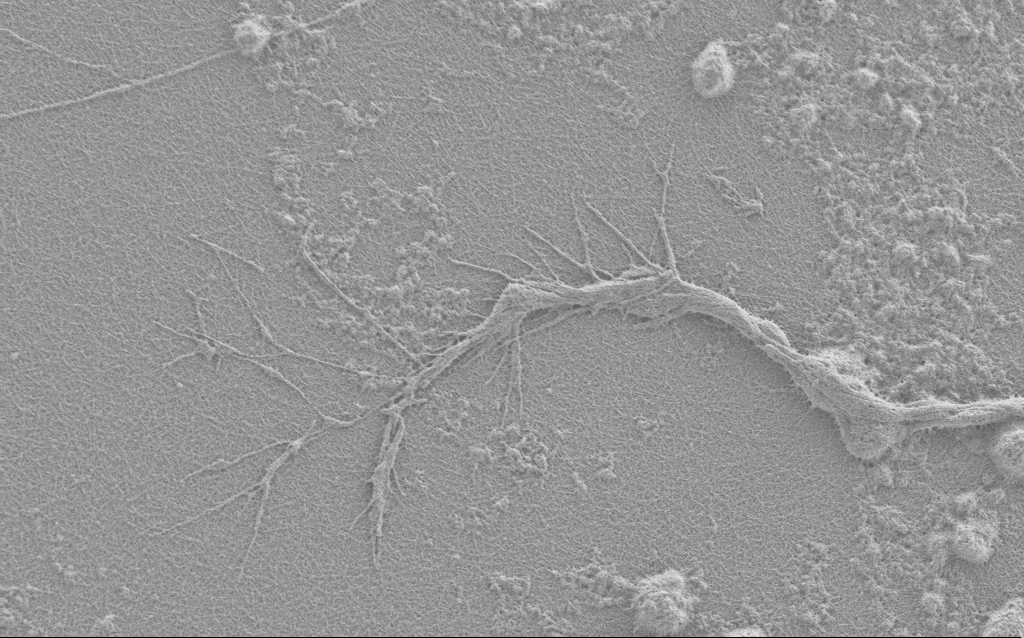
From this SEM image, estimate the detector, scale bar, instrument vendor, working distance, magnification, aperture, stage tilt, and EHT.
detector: SE2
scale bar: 2000 nm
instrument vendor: Zeiss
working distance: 4 mm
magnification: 7.5 K X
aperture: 30 µm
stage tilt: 0°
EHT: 0.9 kV